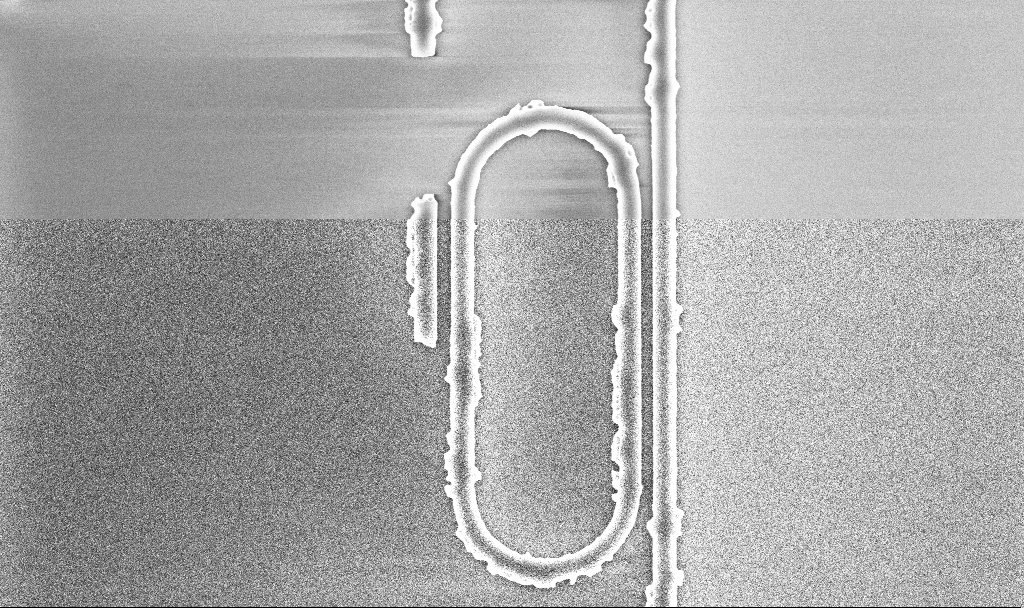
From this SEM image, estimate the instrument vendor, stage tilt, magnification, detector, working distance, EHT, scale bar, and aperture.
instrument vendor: Zeiss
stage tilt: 0°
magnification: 17.8 K X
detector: InLens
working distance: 5.2 mm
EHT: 5 kV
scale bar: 2000 nm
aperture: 30 µm